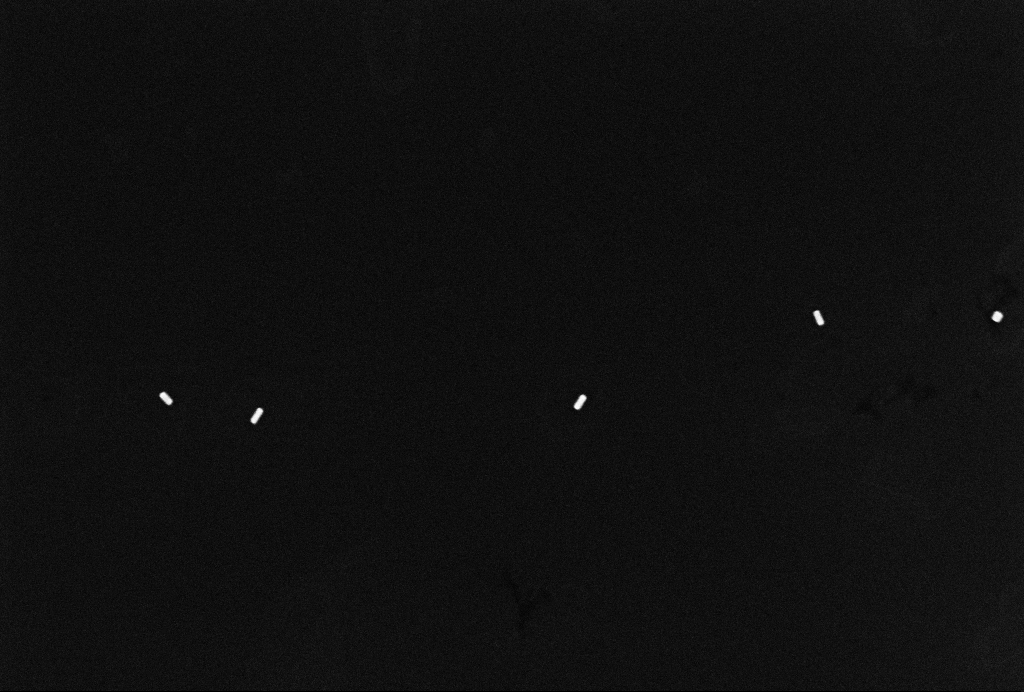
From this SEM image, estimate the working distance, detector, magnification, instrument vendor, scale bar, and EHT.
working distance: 3.2 mm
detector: InLens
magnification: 80 K X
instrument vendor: Zeiss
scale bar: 200 nm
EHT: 10 kV